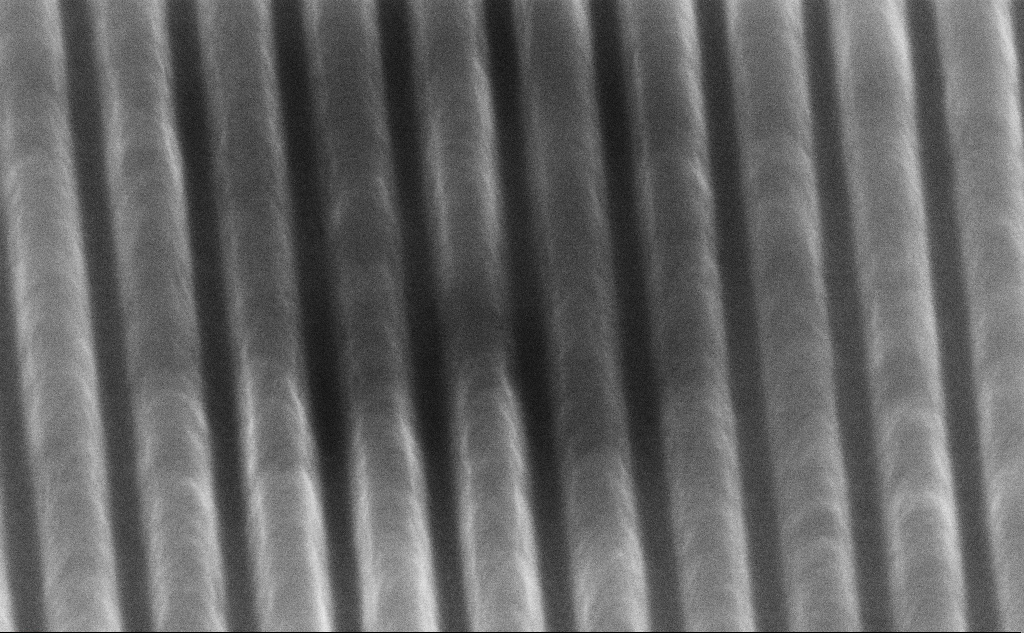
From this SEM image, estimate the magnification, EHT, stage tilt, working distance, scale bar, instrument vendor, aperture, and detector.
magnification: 128.32 K X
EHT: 5 kV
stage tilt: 45°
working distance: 7.4 mm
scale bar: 200 nm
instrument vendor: Zeiss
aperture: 30 µm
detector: InLens